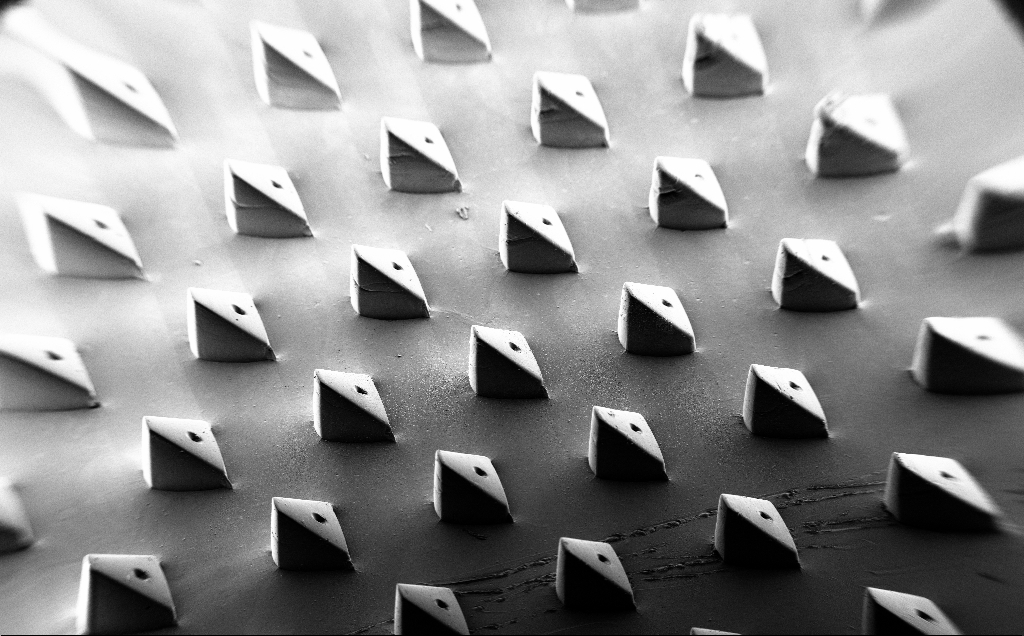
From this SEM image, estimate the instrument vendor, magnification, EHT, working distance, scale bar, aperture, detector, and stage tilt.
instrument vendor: Zeiss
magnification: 0.067 K X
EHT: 5 kV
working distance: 9 mm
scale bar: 1e+06 nm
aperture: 30 µm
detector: SE2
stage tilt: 35°